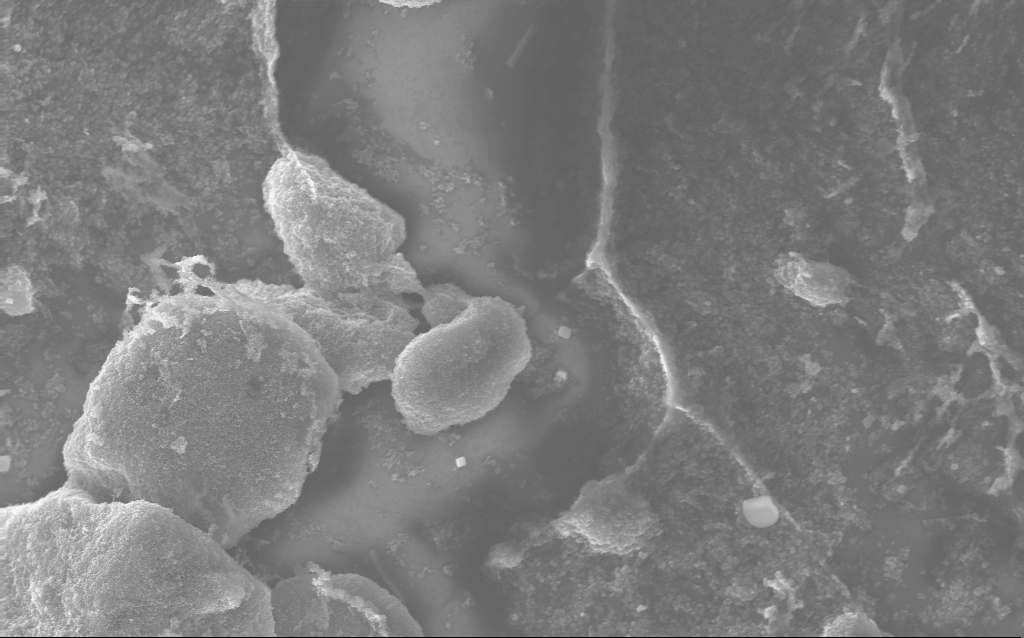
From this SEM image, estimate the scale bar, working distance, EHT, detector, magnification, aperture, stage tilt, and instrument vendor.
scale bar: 1000 nm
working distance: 2.8 mm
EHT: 10 kV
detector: InLens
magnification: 15.33 K X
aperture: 30 µm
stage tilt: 0°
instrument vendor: Zeiss